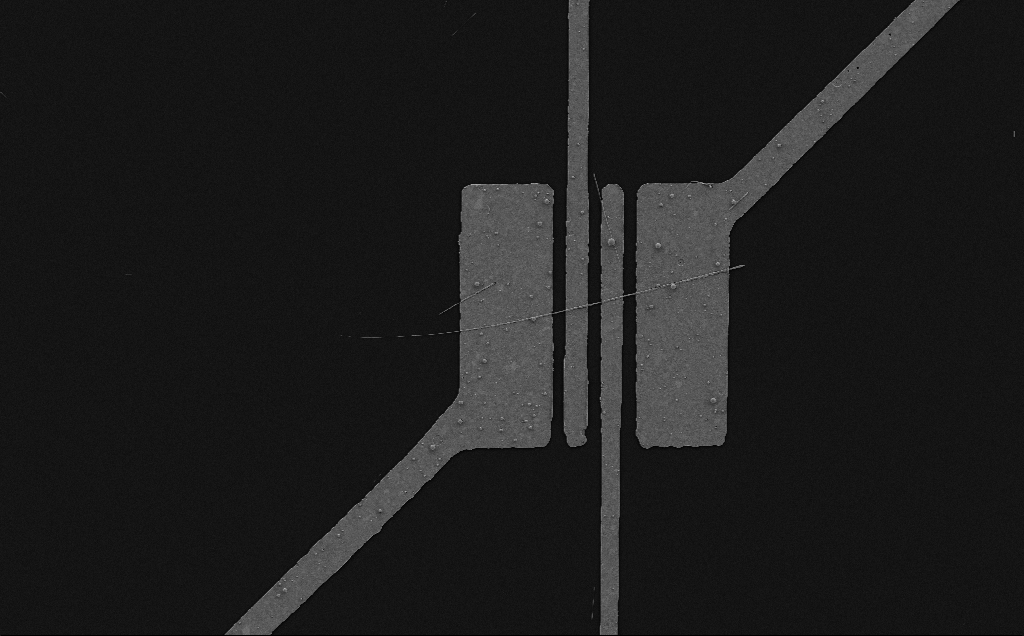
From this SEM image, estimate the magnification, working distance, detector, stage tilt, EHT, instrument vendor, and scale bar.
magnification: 3.28 K X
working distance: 6 mm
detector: SE2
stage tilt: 0°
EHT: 5 kV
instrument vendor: Zeiss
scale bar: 20000 nm